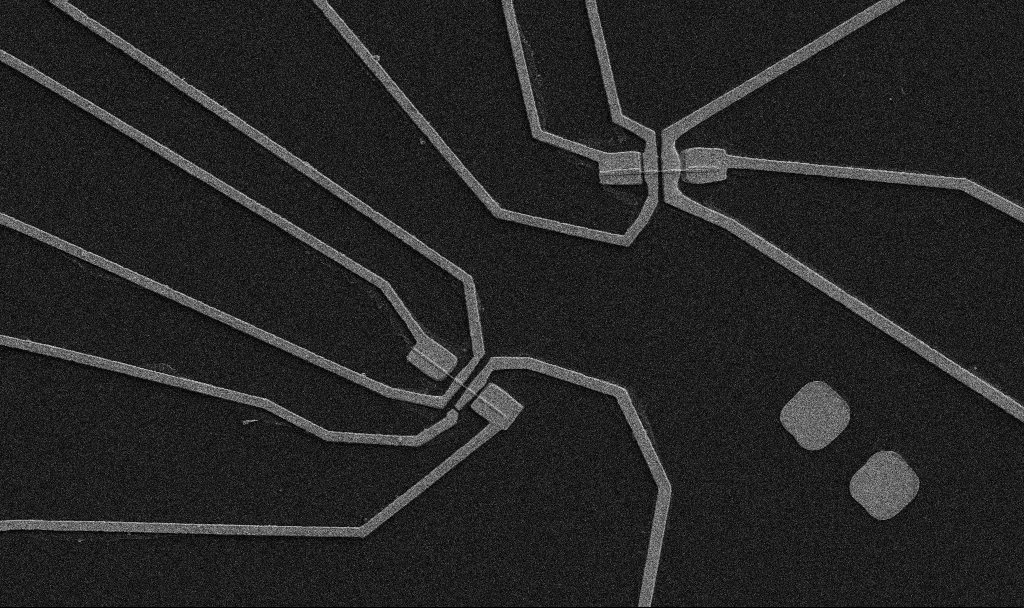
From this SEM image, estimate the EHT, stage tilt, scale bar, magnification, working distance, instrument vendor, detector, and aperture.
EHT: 5 kV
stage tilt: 0°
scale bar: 10000 nm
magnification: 5 K X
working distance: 10.7 mm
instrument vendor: Zeiss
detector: SE2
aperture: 30 µm